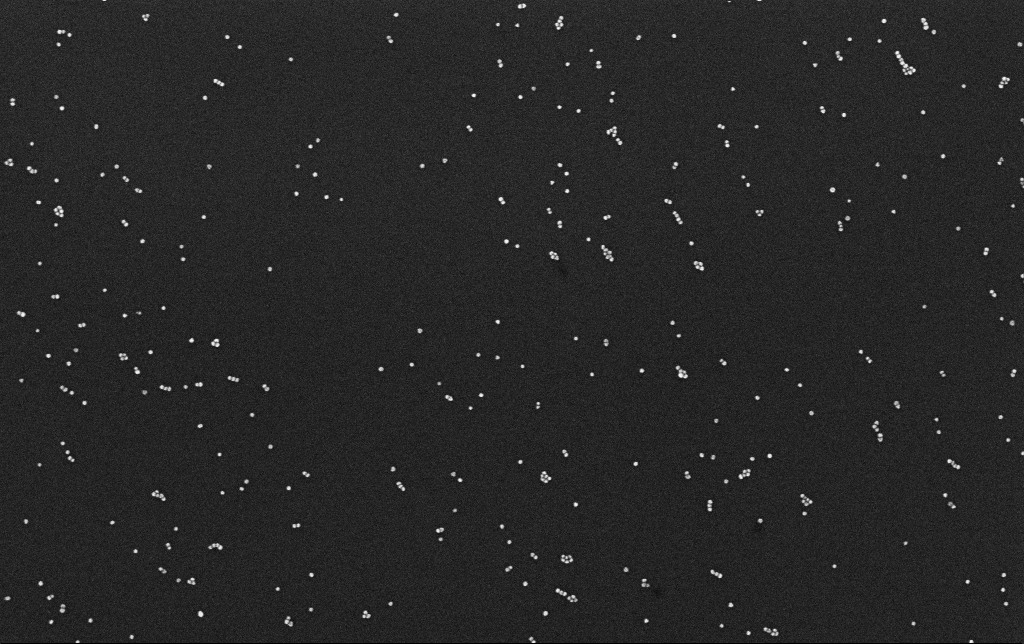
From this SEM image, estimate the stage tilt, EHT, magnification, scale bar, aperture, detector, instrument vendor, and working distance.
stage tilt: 0°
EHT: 10 kV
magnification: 100 K X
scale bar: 200 nm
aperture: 30 µm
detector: InLens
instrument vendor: Zeiss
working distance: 3.1 mm